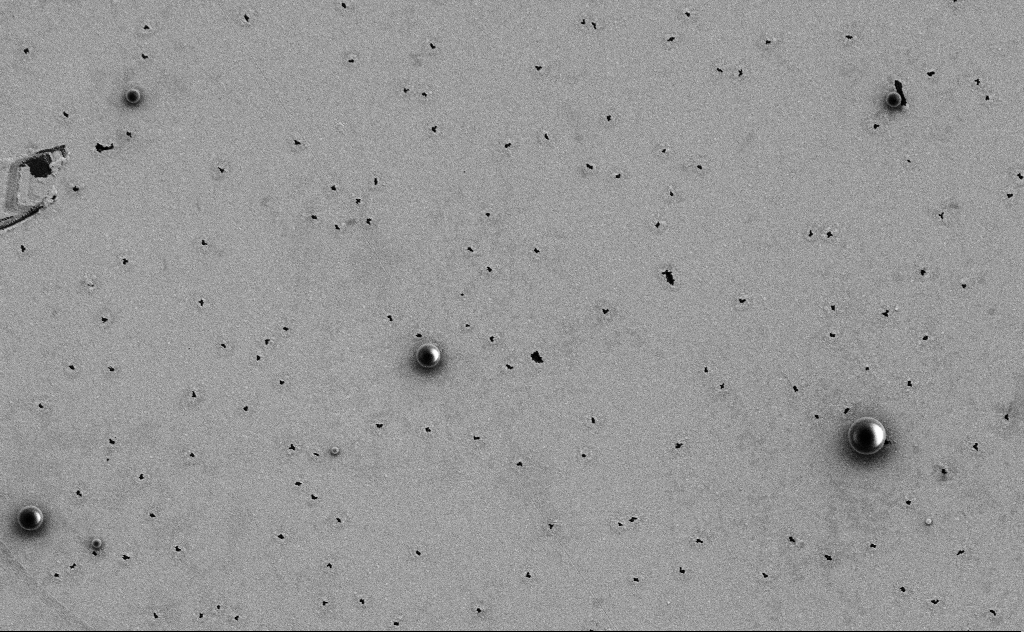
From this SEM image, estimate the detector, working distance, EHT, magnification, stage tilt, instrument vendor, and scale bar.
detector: SE2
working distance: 10 mm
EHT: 3 kV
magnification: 8.2 K X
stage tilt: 0°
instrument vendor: Zeiss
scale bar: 2000 nm